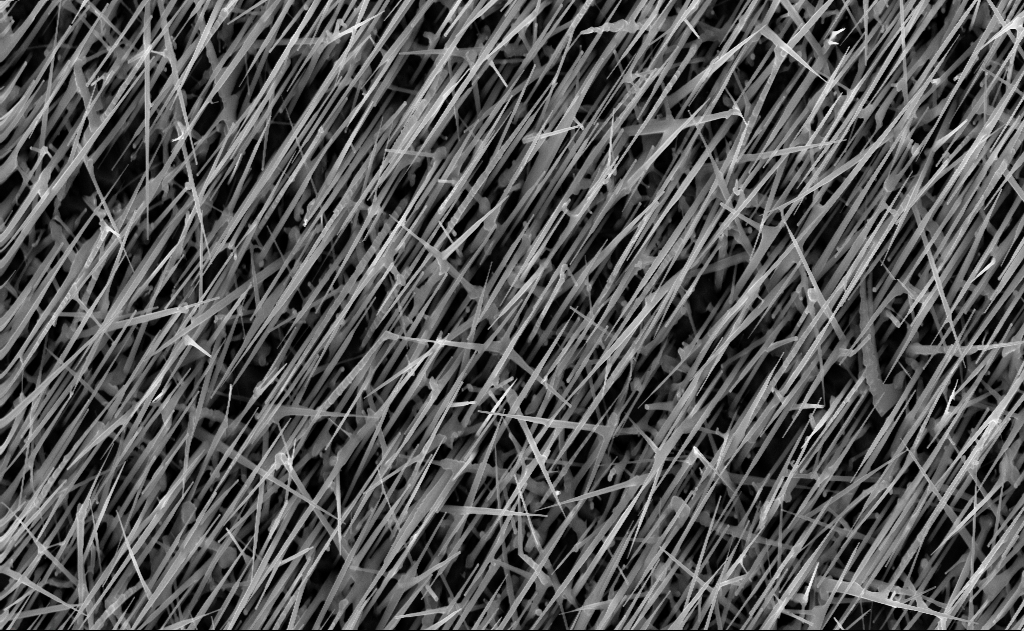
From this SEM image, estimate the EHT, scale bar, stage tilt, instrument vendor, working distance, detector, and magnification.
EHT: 10 kV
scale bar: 2000 nm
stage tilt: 0°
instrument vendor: Zeiss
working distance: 7 mm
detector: InLens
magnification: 20 K X